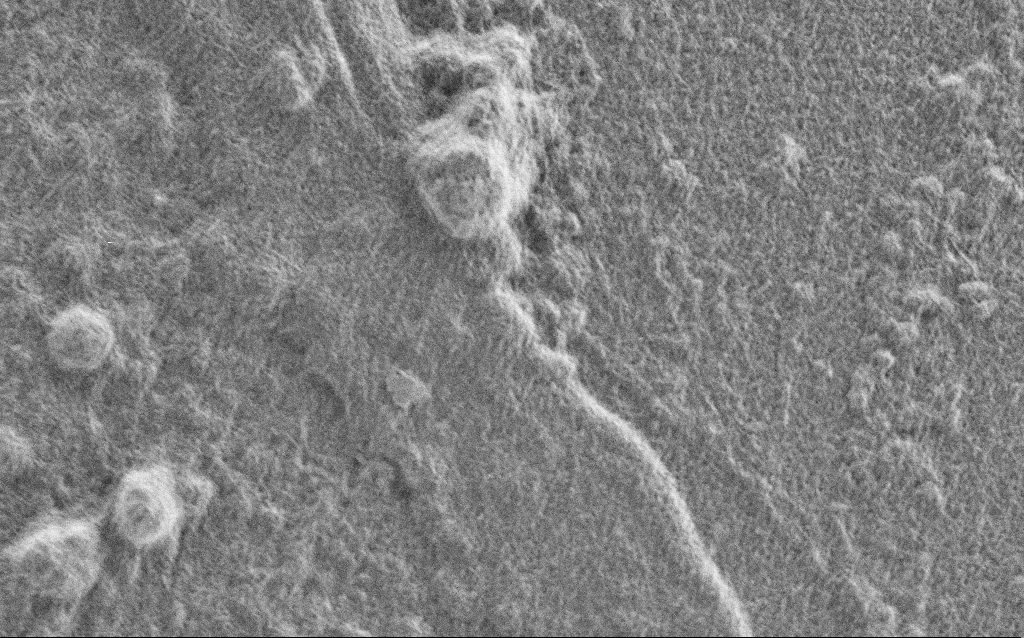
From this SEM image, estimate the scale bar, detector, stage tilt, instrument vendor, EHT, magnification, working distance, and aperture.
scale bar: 2000 nm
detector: SE2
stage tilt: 0°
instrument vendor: Zeiss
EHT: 1 kV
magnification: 10 K X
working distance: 4 mm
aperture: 30 µm